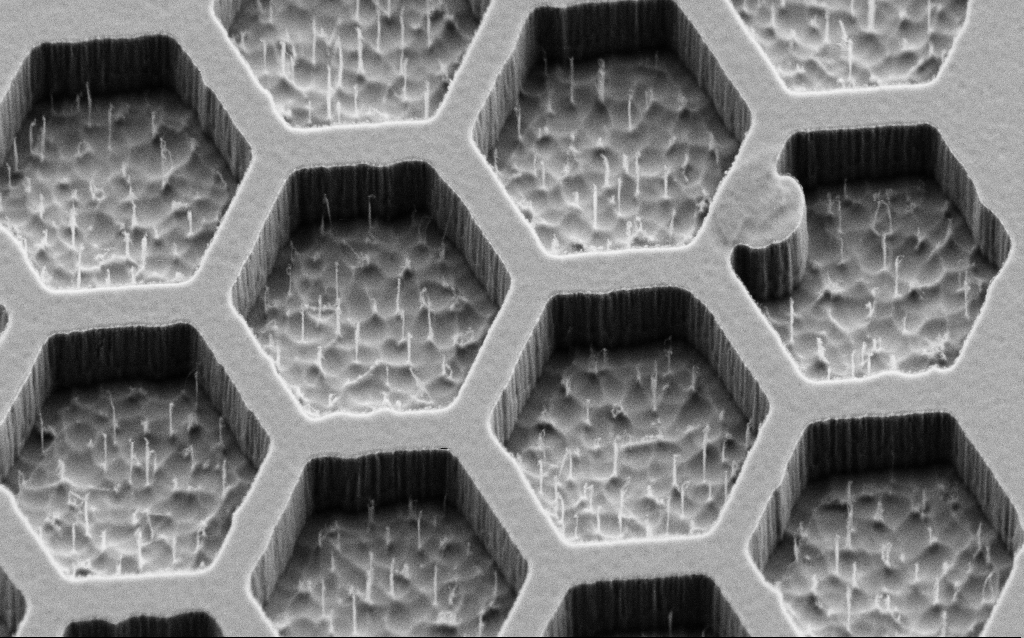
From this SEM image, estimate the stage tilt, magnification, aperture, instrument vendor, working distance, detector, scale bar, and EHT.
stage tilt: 45°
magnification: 34.44 K X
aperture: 30 µm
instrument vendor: Zeiss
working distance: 6 mm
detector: SE2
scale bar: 2000 nm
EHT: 2 kV